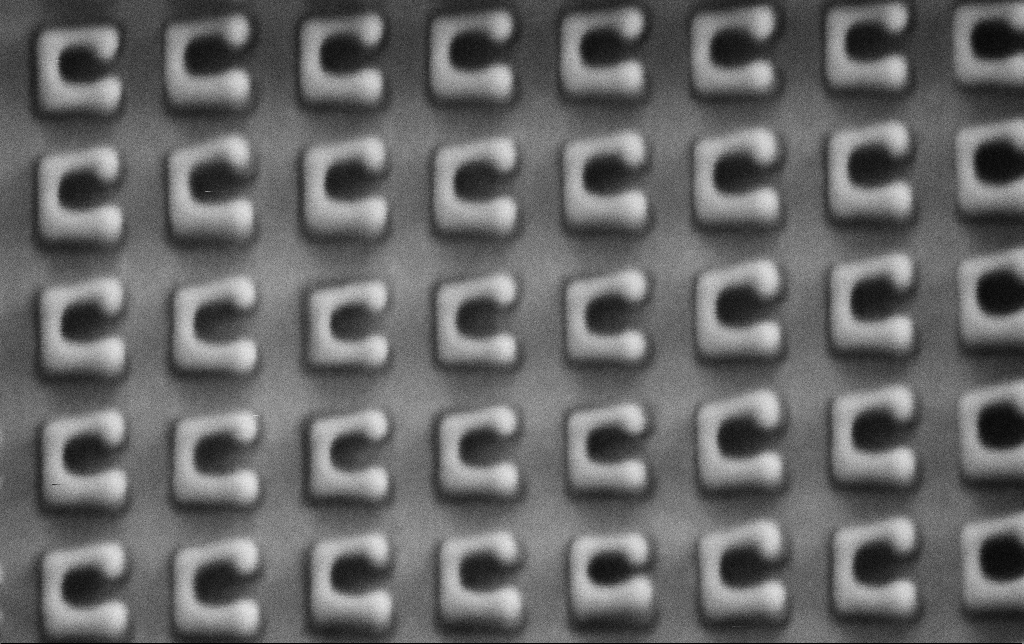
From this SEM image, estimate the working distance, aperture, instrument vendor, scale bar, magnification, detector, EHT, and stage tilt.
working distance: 7.8 mm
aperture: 30 µm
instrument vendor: Zeiss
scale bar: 200 nm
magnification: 103.99 K X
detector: SE2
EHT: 1.5 kV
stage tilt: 0°